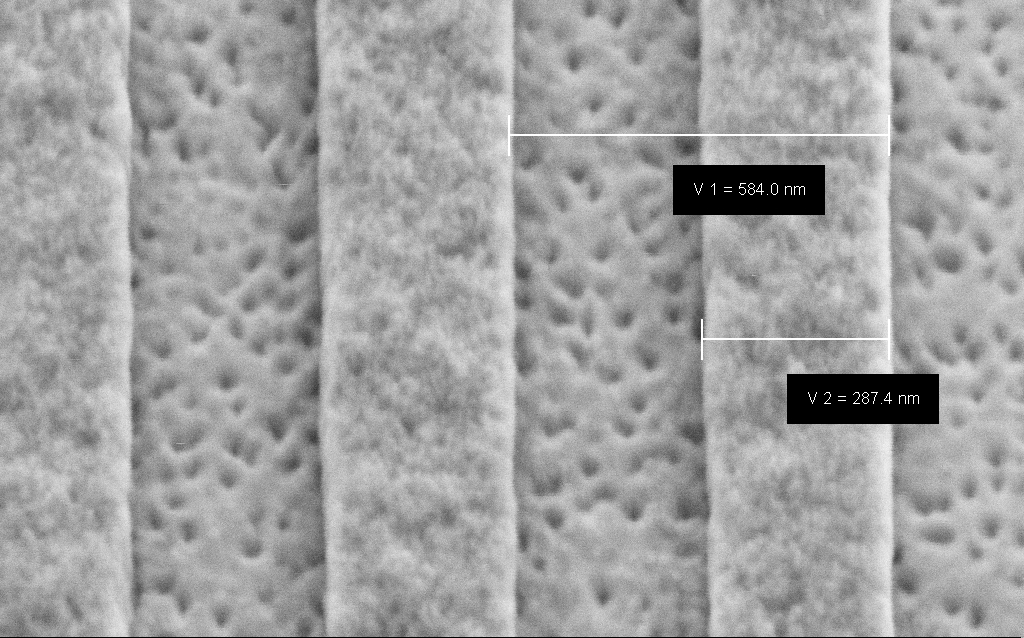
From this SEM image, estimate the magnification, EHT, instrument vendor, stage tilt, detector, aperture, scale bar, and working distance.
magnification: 238.9 K X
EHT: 3 kV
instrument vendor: Zeiss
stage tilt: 45°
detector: SE2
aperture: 30 µm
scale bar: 100 nm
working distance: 6.6 mm